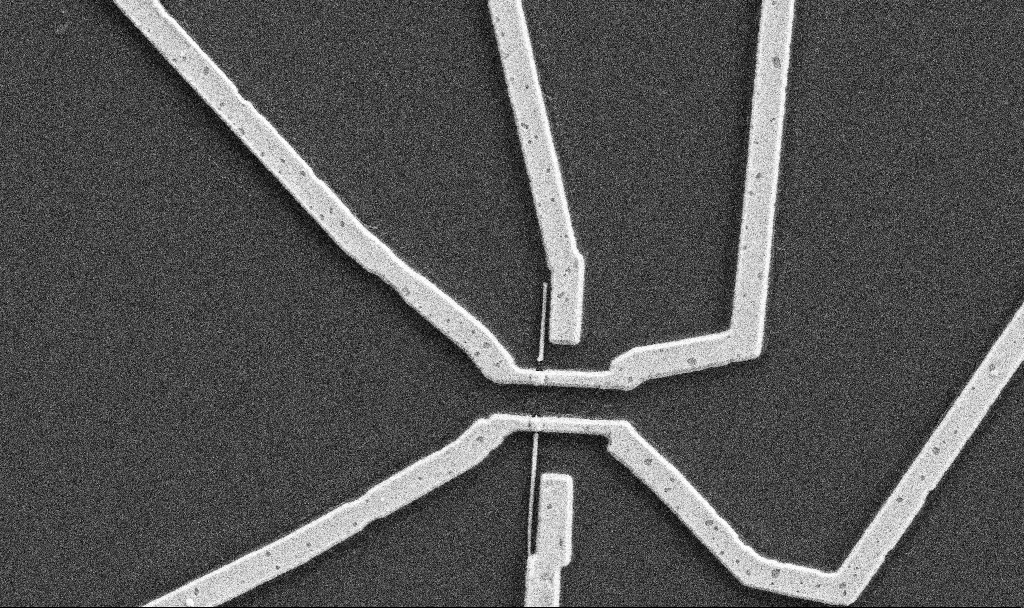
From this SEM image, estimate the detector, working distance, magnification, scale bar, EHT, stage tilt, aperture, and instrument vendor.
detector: SE2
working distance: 10.7 mm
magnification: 16.93 K X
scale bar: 2000 nm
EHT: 5 kV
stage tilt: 0°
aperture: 30 µm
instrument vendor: Zeiss